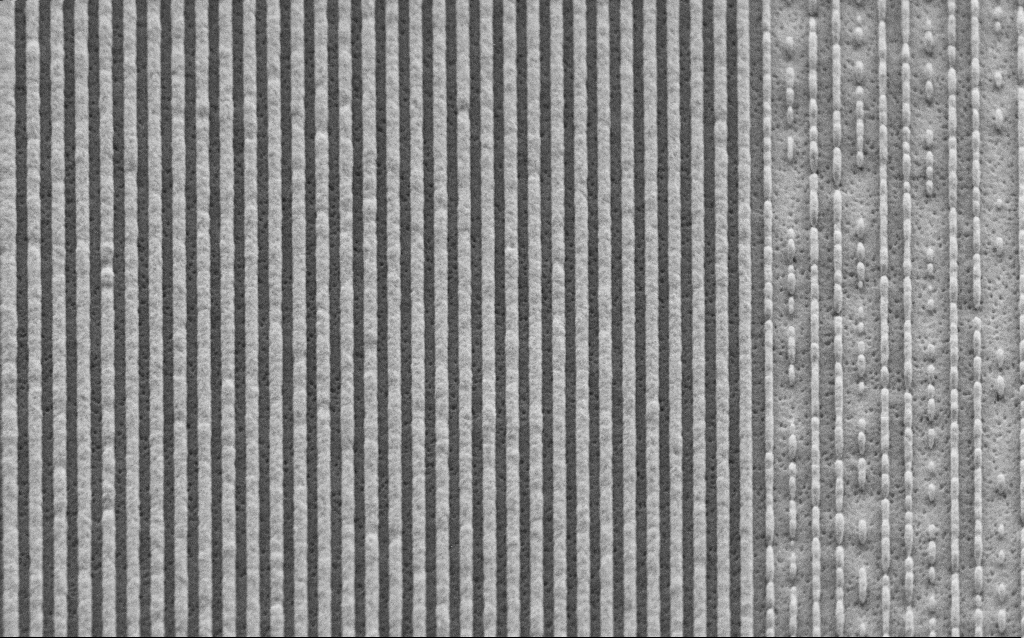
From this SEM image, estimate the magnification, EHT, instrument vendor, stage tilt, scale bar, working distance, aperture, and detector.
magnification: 42.97 K X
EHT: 3 kV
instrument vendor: Zeiss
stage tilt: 45°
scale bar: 1000 nm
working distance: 6.6 mm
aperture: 30 µm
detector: SE2